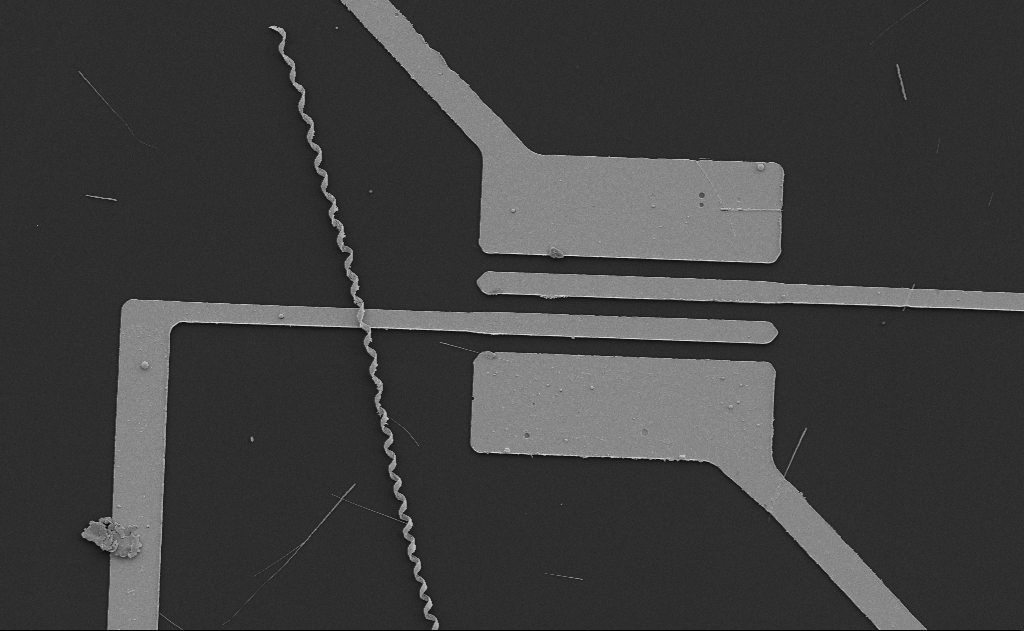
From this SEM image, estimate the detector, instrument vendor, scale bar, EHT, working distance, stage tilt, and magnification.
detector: SE2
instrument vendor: Zeiss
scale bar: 10000 nm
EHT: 5 kV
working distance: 13 mm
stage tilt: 0°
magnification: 3.7 K X